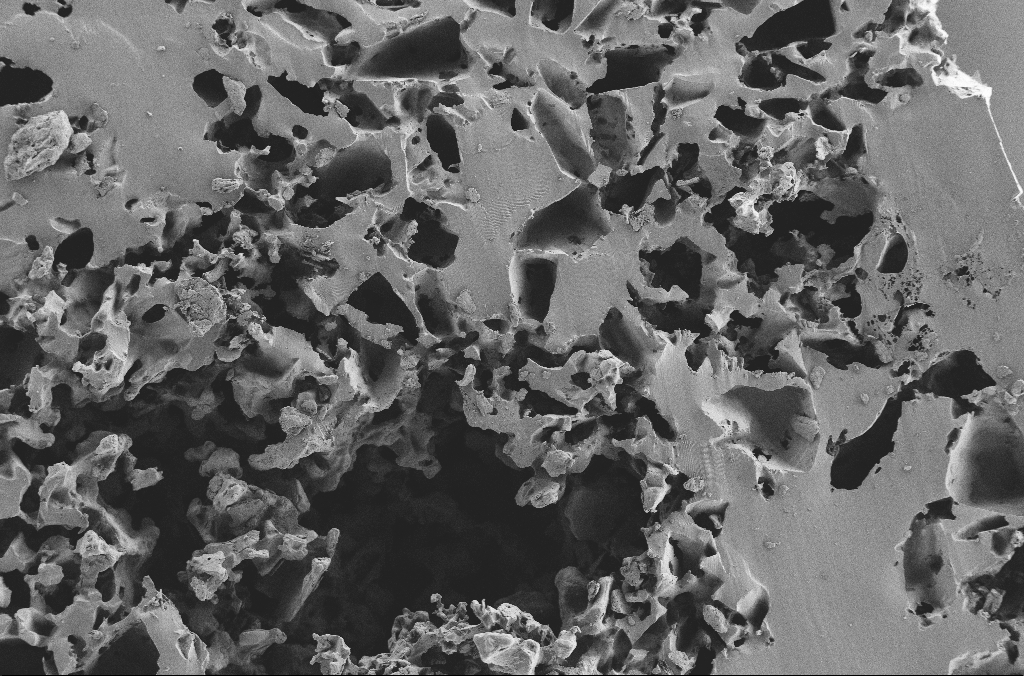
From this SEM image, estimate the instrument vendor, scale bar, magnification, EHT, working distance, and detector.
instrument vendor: Zeiss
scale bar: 100000 nm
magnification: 0.25 K X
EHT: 2 kV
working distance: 3.1 mm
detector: SE2